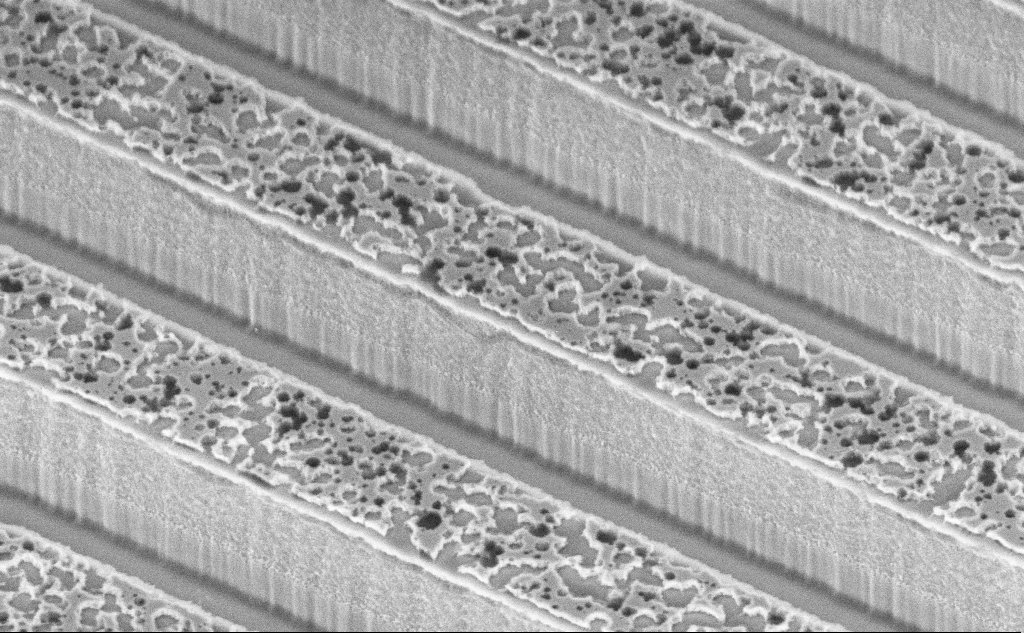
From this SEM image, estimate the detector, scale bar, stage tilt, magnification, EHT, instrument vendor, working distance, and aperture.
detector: InLens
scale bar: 2000 nm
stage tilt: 45°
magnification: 30.53 K X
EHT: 3 kV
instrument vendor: Zeiss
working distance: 12.8 mm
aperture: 30 µm